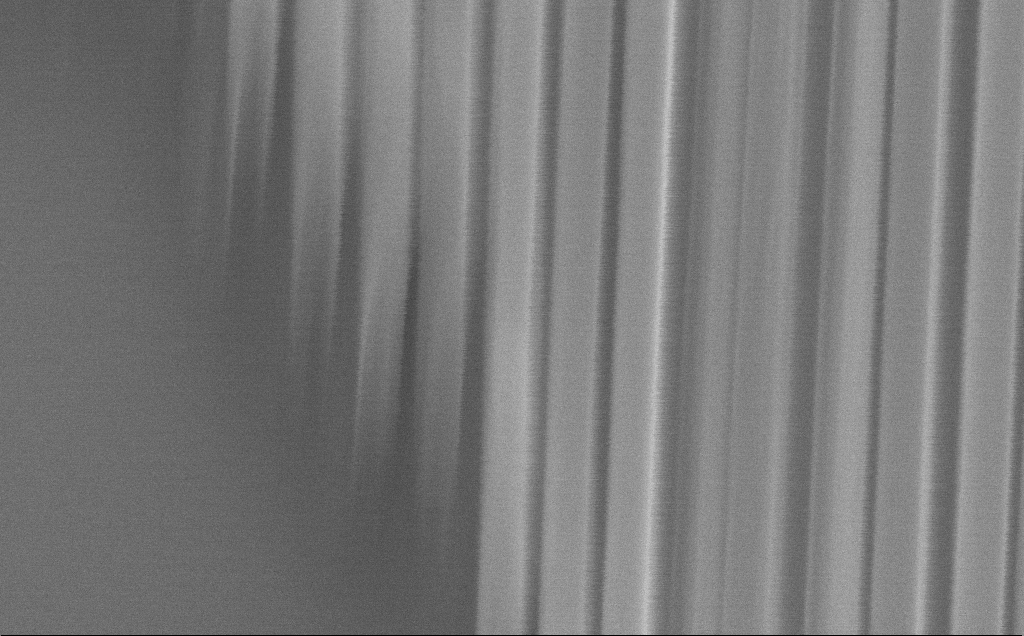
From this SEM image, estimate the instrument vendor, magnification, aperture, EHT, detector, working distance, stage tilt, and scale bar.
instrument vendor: Zeiss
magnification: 140.58 K X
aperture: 30 µm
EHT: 10 kV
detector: SE2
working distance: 10 mm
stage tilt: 45°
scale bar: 200 nm